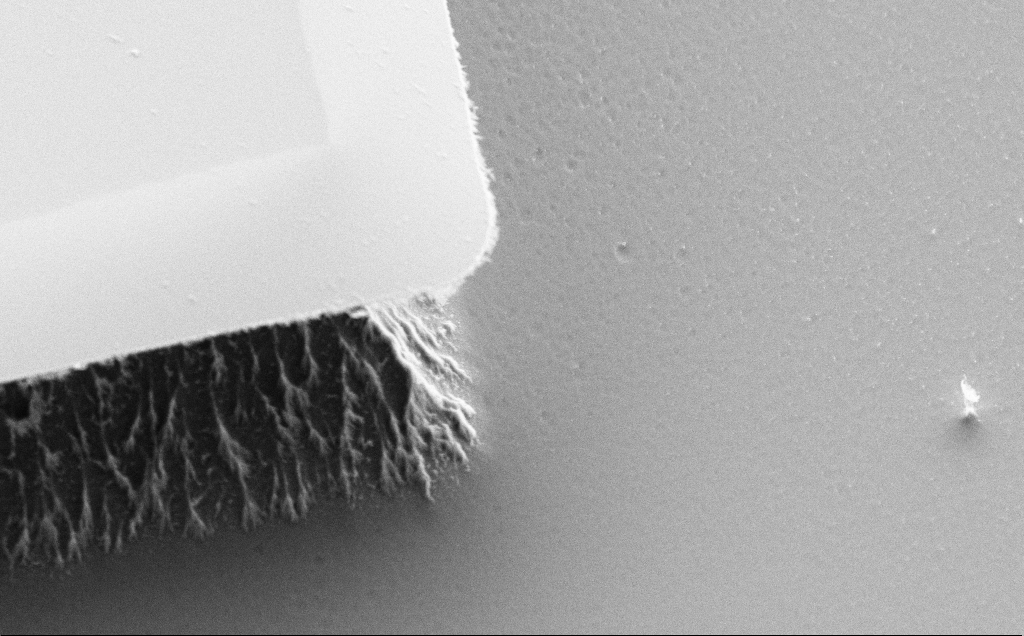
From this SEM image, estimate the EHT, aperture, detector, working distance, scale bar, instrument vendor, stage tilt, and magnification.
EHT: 5 kV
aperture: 30 µm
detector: SE2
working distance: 8 mm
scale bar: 2000 nm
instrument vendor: Zeiss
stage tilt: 45°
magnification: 7.81 K X